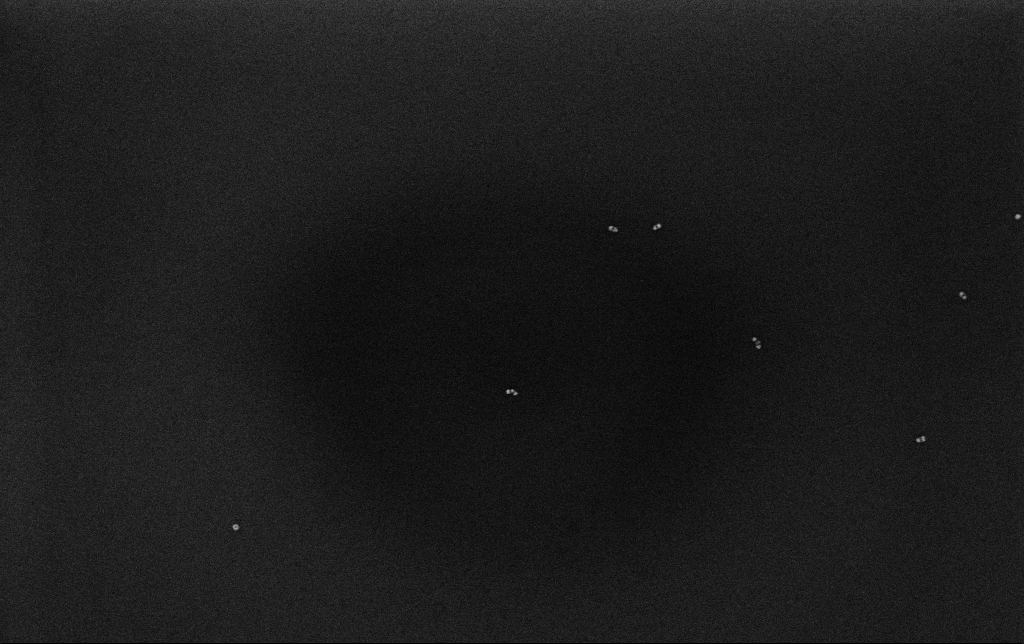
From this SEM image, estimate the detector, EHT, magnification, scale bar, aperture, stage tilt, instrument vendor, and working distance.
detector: InLens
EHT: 10 kV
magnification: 100 K X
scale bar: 200 nm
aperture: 30 µm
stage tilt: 0°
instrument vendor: Zeiss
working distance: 3.2 mm